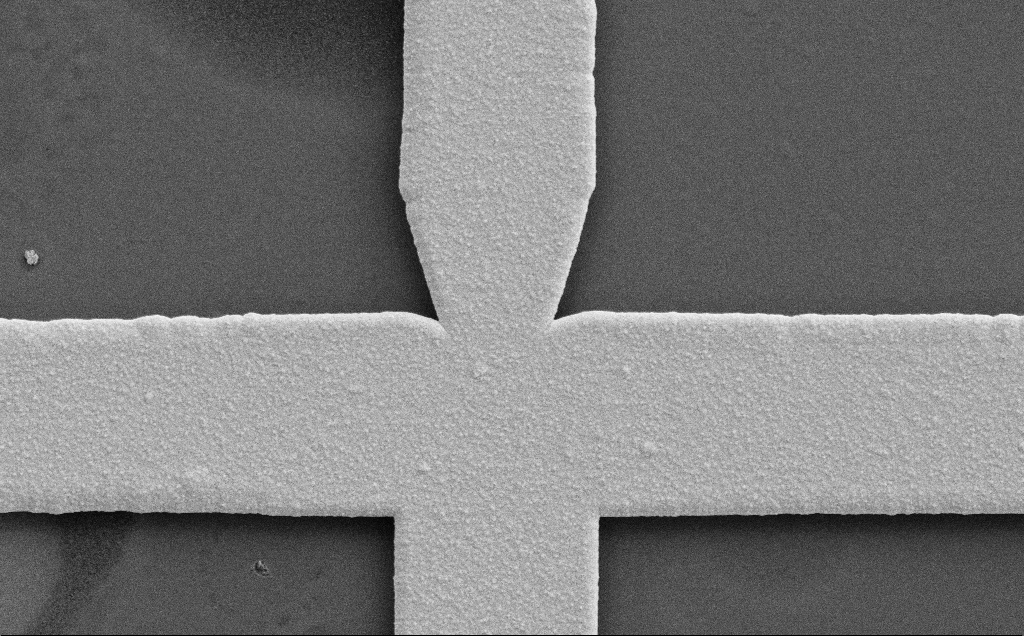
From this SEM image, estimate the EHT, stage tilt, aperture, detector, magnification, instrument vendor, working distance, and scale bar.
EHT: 10 kV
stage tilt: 0°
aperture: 30 µm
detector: SE2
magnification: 6.46 K X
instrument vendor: Zeiss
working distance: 12 mm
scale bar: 10000 nm